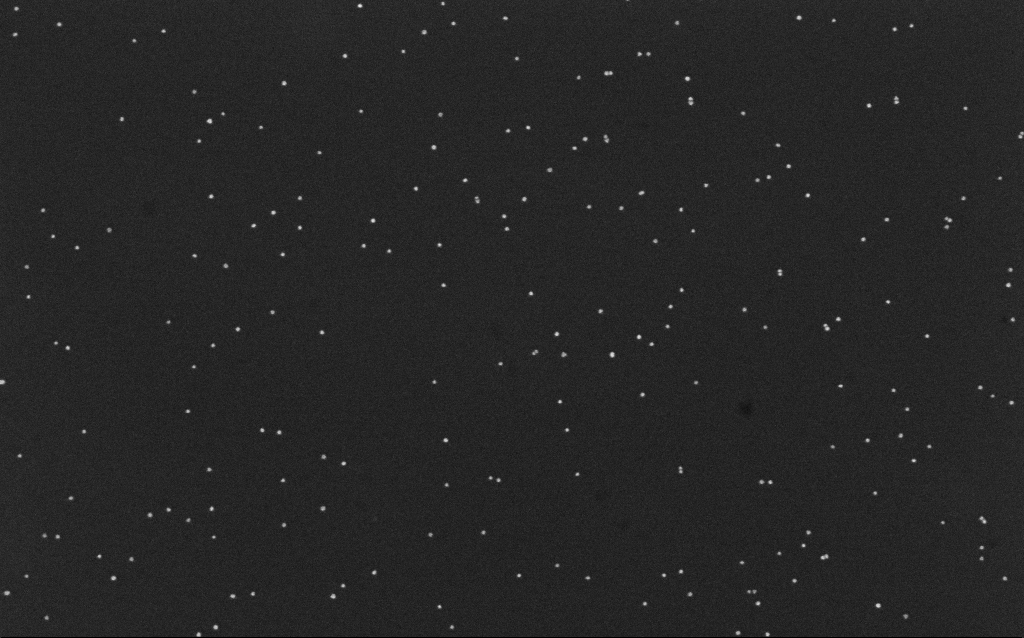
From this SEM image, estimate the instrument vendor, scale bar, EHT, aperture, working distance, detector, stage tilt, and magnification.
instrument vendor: Zeiss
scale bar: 200 nm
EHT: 10 kV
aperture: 30 µm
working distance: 6.5 mm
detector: InLens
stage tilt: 0°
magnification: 100 K X